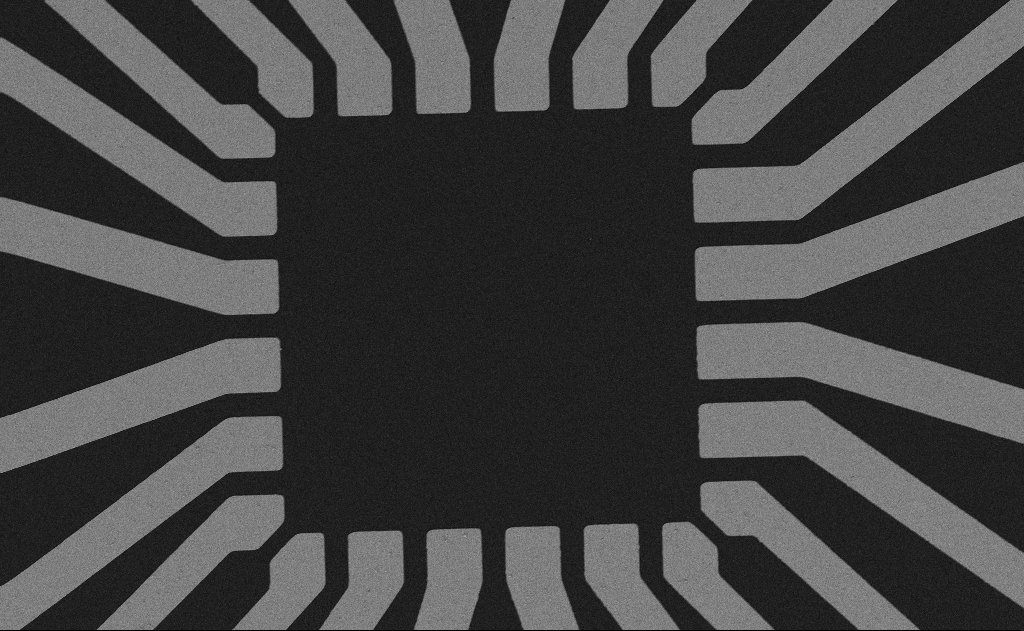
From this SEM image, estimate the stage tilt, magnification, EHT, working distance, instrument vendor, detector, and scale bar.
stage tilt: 0°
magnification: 0.965 K X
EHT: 5 kV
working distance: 7 mm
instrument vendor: Zeiss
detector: SE2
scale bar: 20000 nm